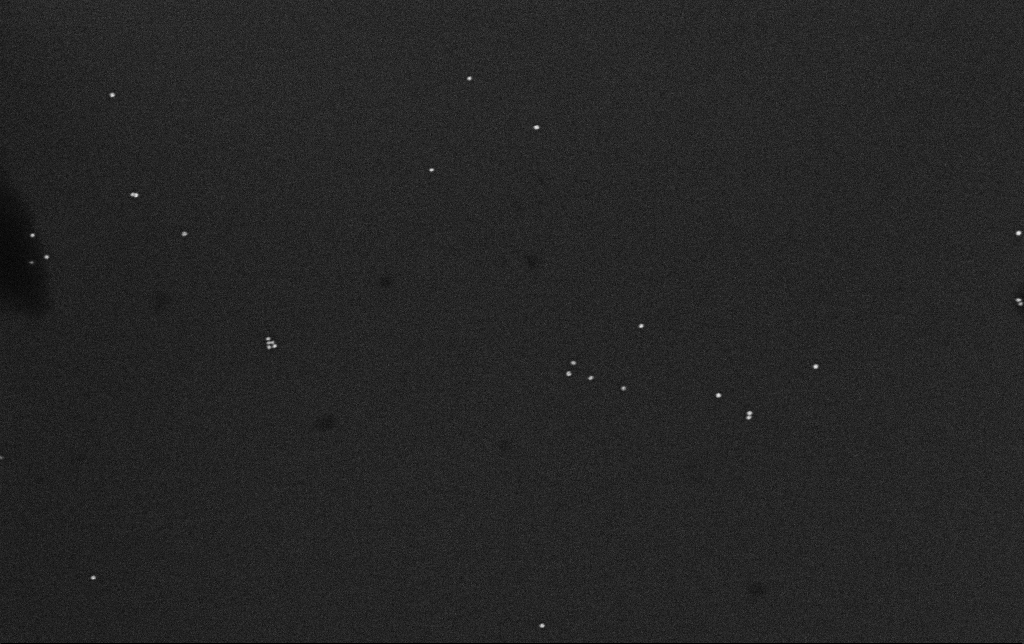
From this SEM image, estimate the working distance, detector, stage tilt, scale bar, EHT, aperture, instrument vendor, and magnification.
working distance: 3.2 mm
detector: InLens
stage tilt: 0°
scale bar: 200 nm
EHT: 10 kV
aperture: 30 µm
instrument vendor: Zeiss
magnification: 100 K X